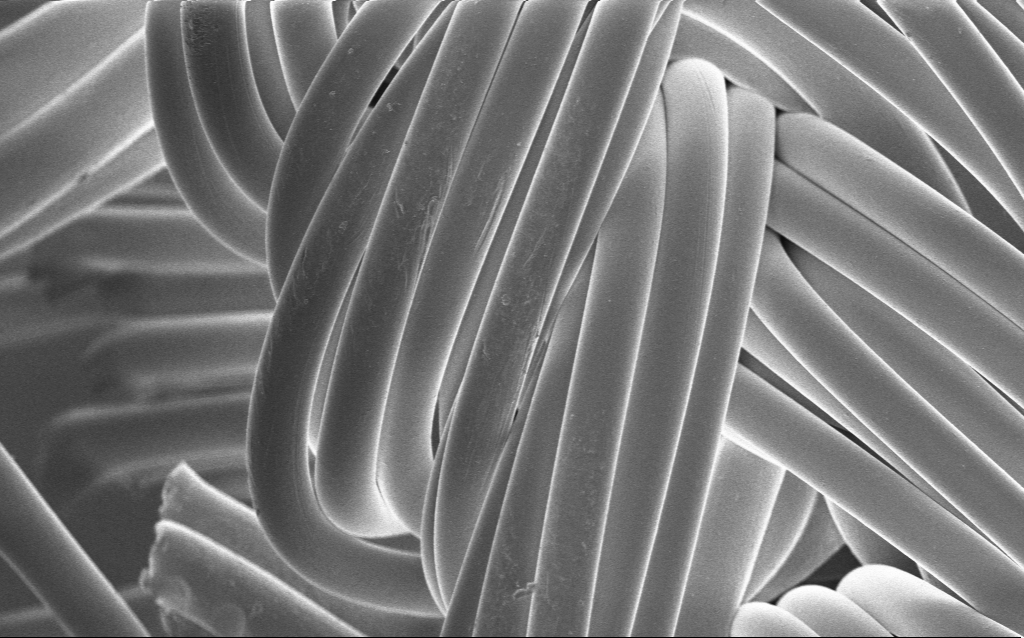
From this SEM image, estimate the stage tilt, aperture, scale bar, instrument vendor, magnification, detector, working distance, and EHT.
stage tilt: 0°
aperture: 30 µm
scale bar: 20000 nm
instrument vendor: Zeiss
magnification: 0.927 K X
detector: InLens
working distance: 4 mm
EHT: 1 kV